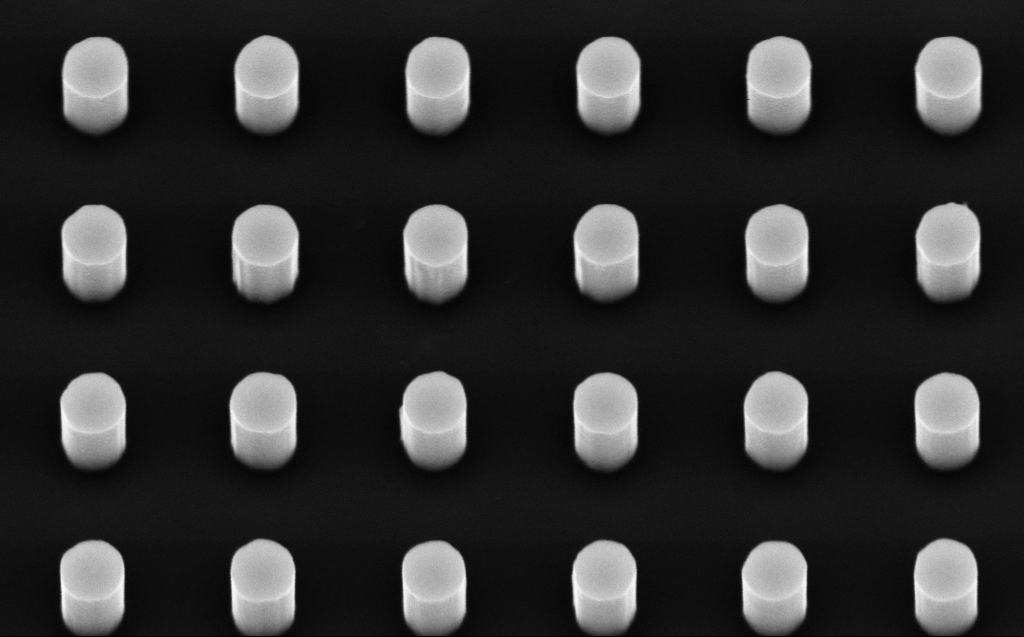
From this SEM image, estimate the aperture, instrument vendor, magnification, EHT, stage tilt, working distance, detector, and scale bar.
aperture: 30 µm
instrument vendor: Zeiss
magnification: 60 K X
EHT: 5 kV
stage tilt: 30°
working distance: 6 mm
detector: InLens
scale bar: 1000 nm